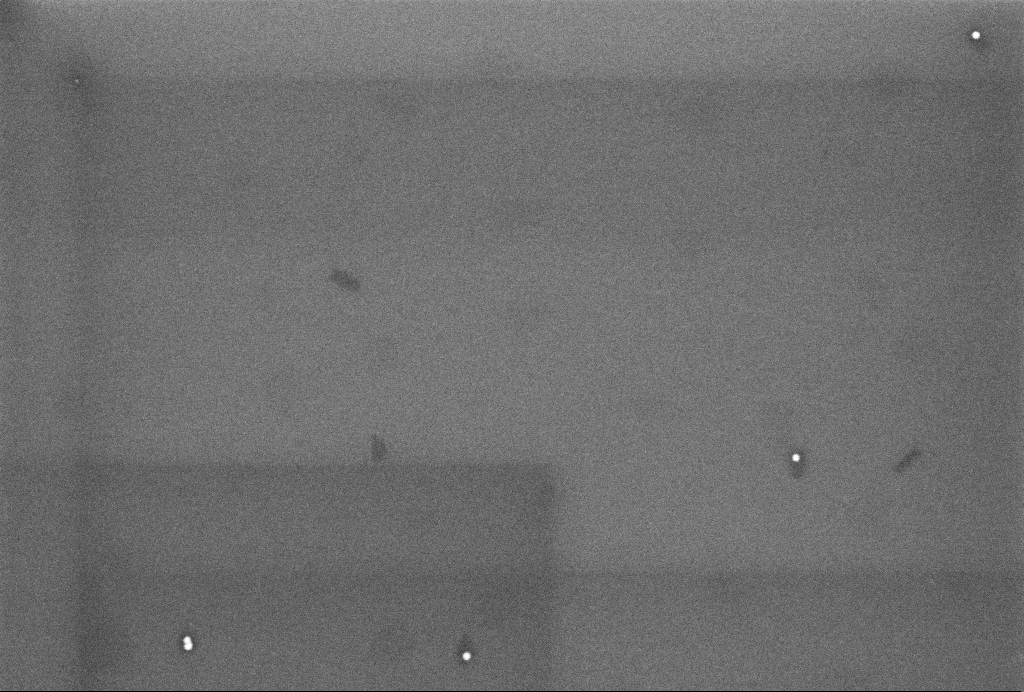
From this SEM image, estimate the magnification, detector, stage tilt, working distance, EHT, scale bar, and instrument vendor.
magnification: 102.77 K X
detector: InLens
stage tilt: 0°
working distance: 3.2 mm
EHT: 3 kV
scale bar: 200 nm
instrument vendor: Zeiss